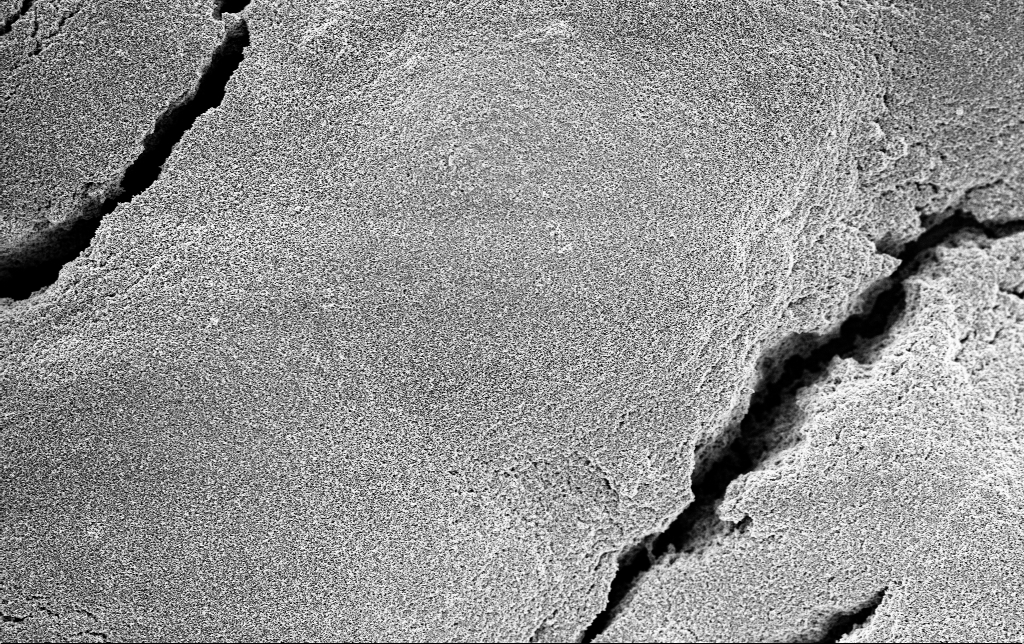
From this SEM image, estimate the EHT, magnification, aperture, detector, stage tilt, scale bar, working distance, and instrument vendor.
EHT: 3 kV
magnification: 5 K X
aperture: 30 µm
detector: InLens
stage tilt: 0°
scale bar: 10000 nm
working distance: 2.8 mm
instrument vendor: Zeiss